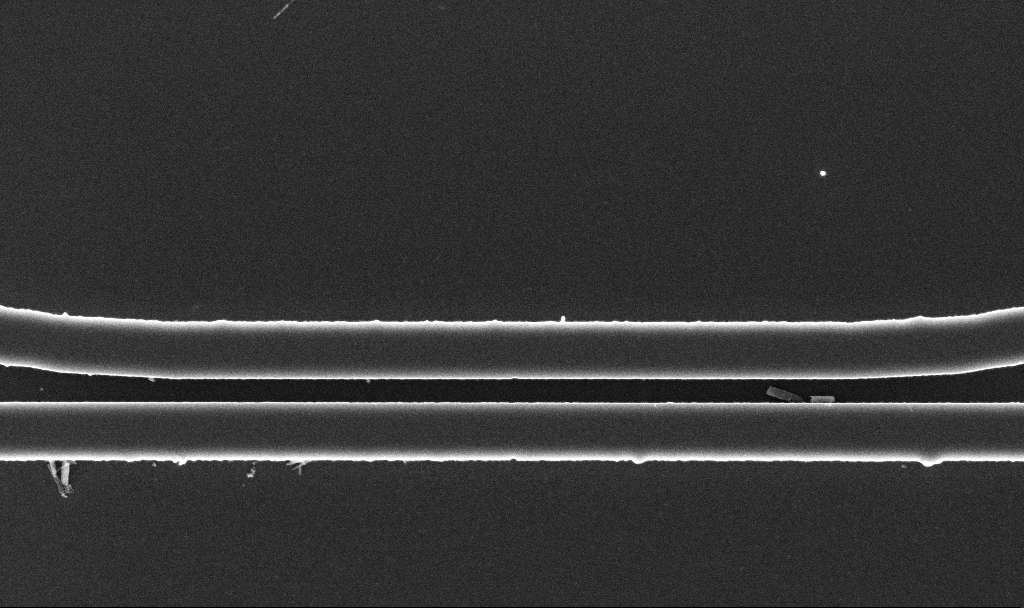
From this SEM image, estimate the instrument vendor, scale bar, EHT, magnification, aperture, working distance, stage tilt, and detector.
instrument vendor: Zeiss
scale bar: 1000 nm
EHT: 3 kV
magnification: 42.04 K X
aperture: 30 µm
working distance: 2.9 mm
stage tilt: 0°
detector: InLens